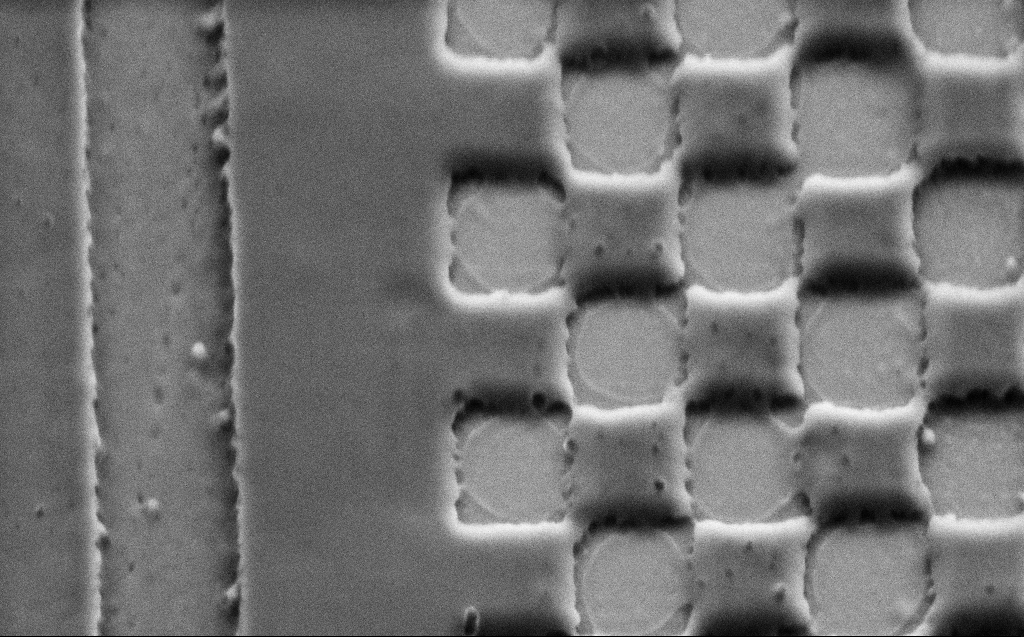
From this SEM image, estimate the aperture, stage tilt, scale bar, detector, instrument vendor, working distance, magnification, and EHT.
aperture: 30 µm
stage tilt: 45°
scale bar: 1000 nm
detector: SE2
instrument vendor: Zeiss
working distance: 6 mm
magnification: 43.29 K X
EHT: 3 kV